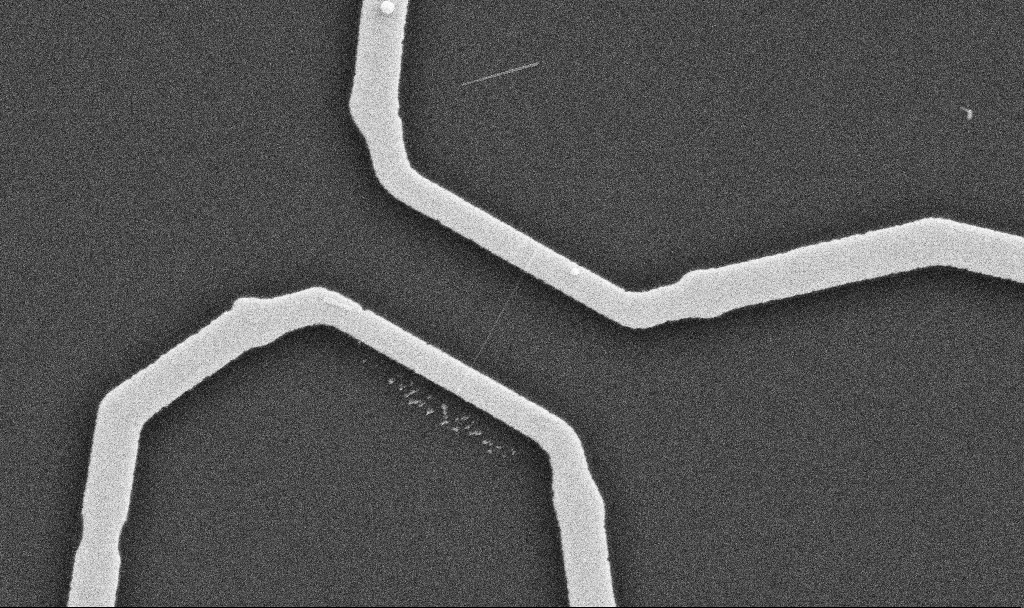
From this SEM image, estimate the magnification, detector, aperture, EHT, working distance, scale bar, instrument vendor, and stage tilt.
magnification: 20 K X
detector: SE2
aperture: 30 µm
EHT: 10 kV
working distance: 10.7 mm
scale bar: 2000 nm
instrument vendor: Zeiss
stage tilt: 0°